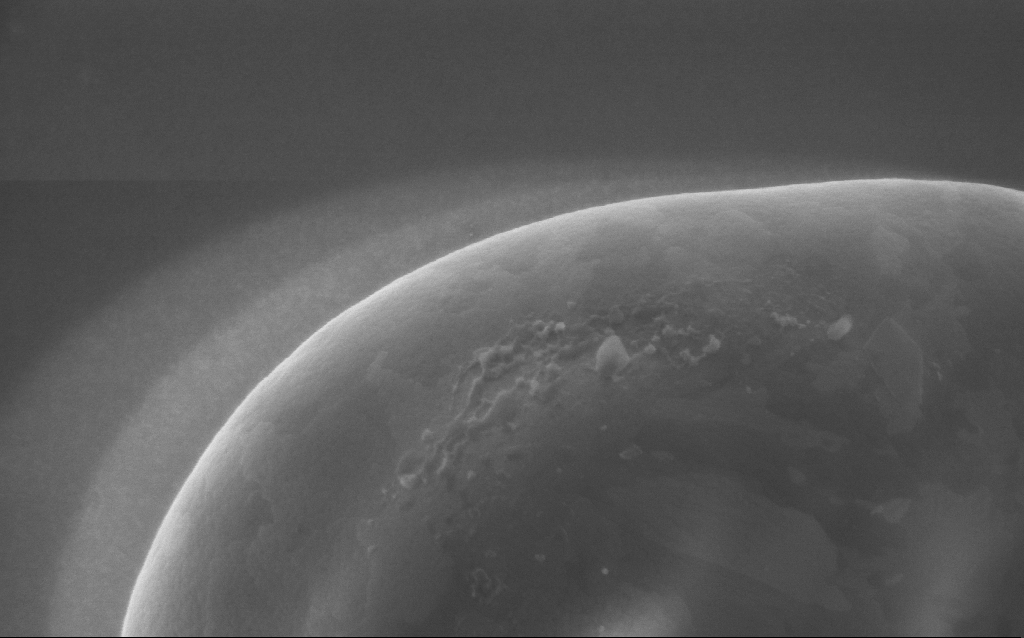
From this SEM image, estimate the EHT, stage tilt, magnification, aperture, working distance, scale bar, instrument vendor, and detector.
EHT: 5 kV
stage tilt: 0°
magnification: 100 K X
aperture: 30 µm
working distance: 4 mm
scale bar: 200 nm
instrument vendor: Zeiss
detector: InLens